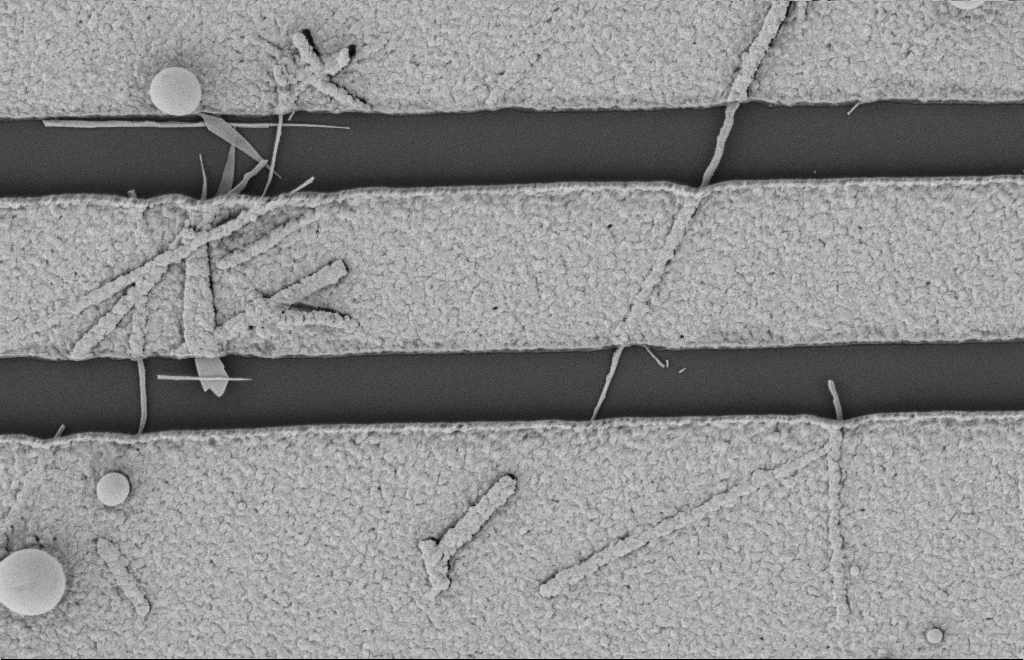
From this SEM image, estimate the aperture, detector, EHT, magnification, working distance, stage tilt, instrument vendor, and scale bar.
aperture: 20 µm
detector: SE2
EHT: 2 kV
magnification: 21.99 K X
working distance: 12 mm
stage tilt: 0°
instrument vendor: Zeiss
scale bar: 2000 nm